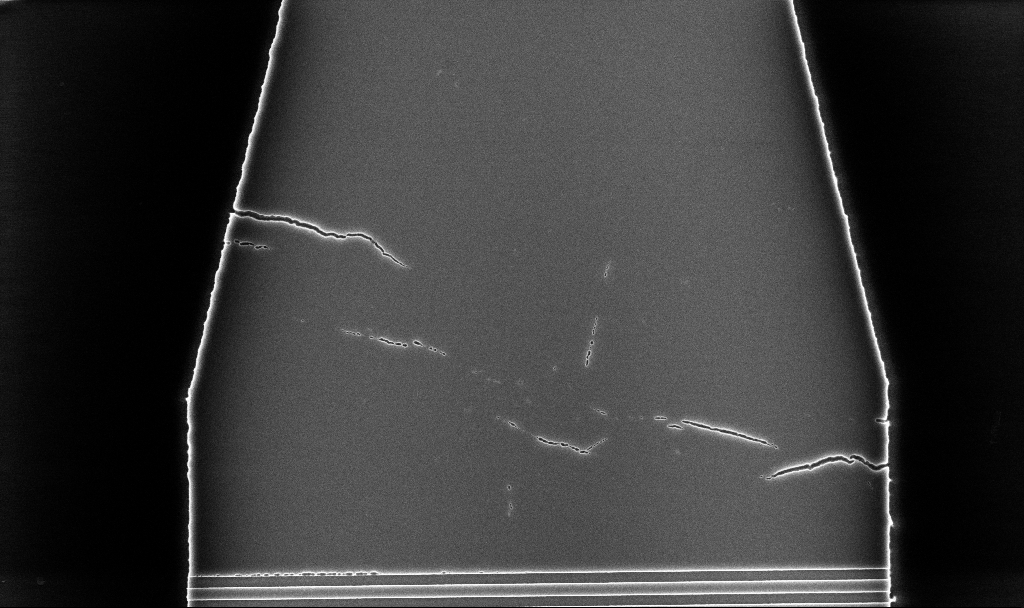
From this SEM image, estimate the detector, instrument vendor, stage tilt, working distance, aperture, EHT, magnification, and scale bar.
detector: InLens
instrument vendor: Zeiss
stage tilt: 0°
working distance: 5.2 mm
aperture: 30 µm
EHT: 5 kV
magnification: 13.08 K X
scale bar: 2000 nm